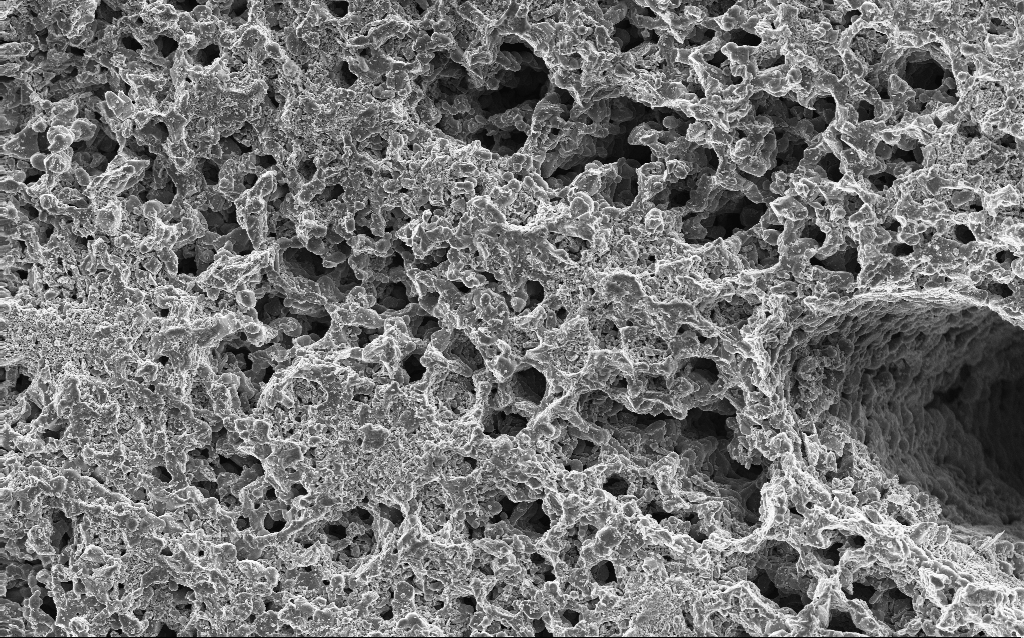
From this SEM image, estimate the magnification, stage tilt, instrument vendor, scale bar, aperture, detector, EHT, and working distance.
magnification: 1 K X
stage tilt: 0°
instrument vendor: Zeiss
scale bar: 20000 nm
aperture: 30 µm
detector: InLens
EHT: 10 kV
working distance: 3 mm